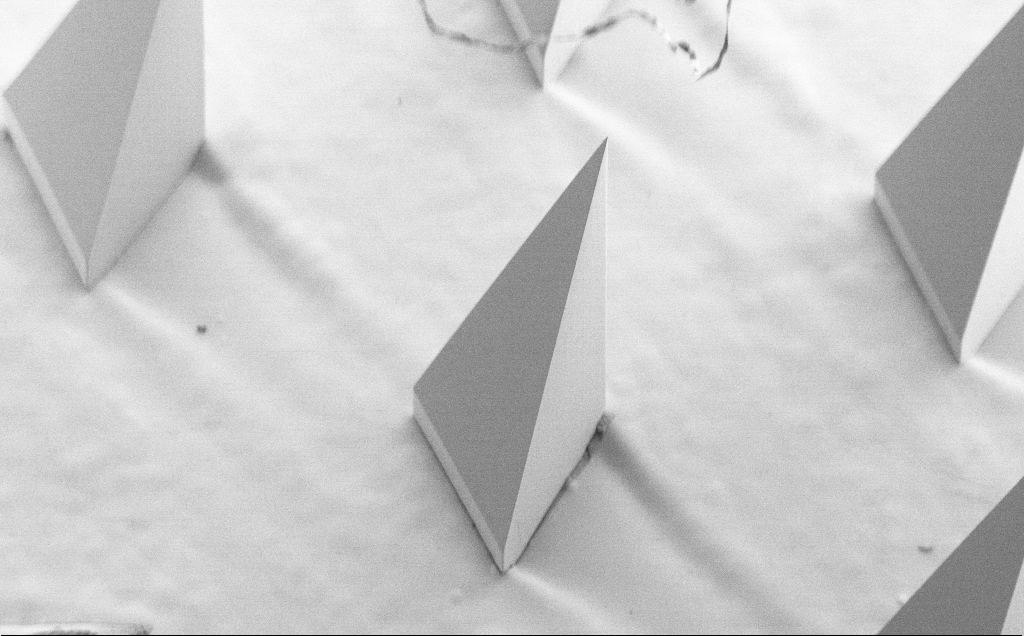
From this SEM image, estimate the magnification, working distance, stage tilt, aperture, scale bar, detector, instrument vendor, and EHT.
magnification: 0.121 K X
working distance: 8 mm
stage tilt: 40°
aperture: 30 µm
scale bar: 200000 nm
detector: SE2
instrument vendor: Zeiss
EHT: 5 kV